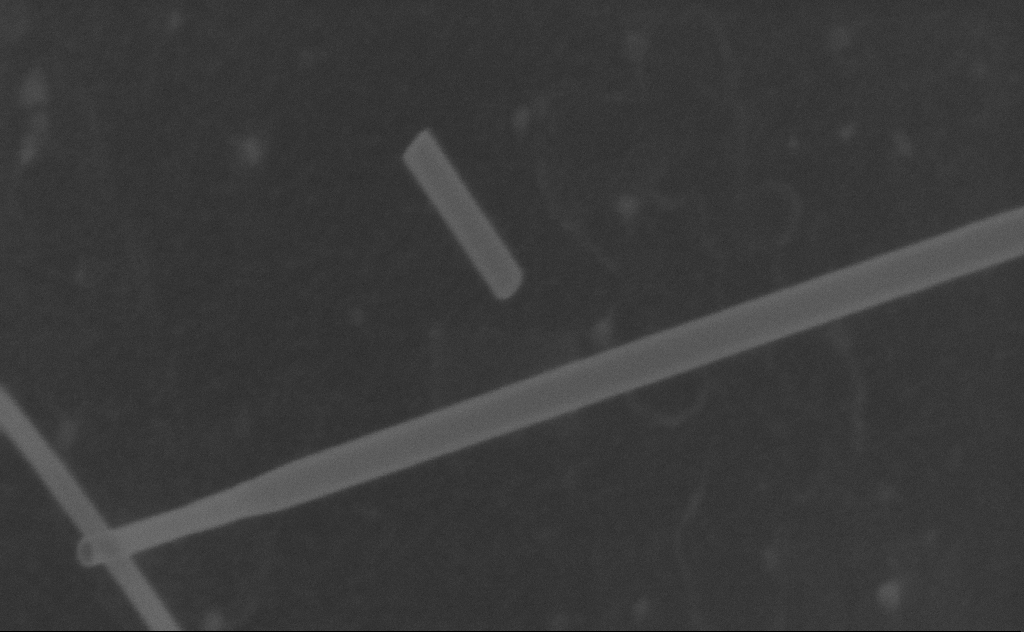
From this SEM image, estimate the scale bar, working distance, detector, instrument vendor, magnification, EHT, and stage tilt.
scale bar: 100 nm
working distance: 7 mm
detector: SE2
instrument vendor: Zeiss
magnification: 362.93 K X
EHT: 10 kV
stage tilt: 0°